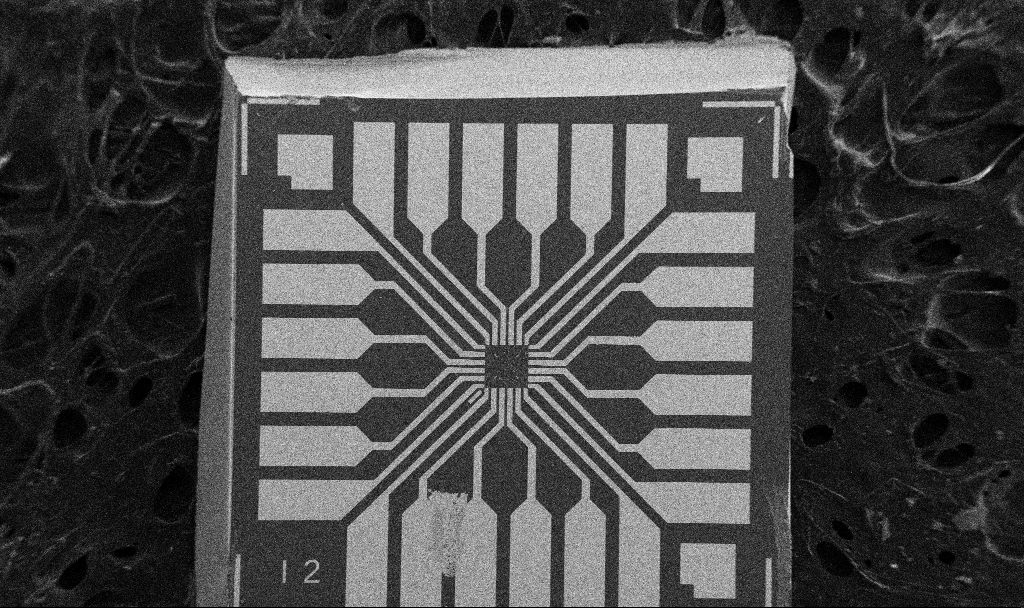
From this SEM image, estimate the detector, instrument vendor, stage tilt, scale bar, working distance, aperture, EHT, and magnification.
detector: SE2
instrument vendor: Zeiss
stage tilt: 0°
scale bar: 200000 nm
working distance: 10.7 mm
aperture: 30 µm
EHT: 5 kV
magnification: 0.1 K X